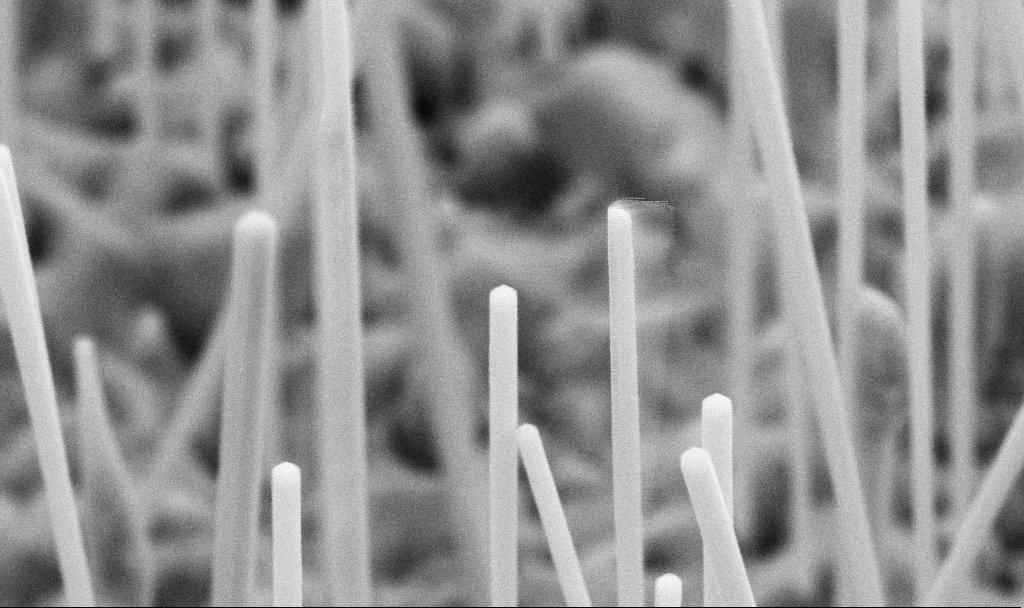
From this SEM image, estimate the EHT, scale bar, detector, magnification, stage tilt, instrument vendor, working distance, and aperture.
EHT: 5 kV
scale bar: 200 nm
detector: SE2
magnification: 82.25 K X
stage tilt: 45°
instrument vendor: Zeiss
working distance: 7.2 mm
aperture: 30 µm